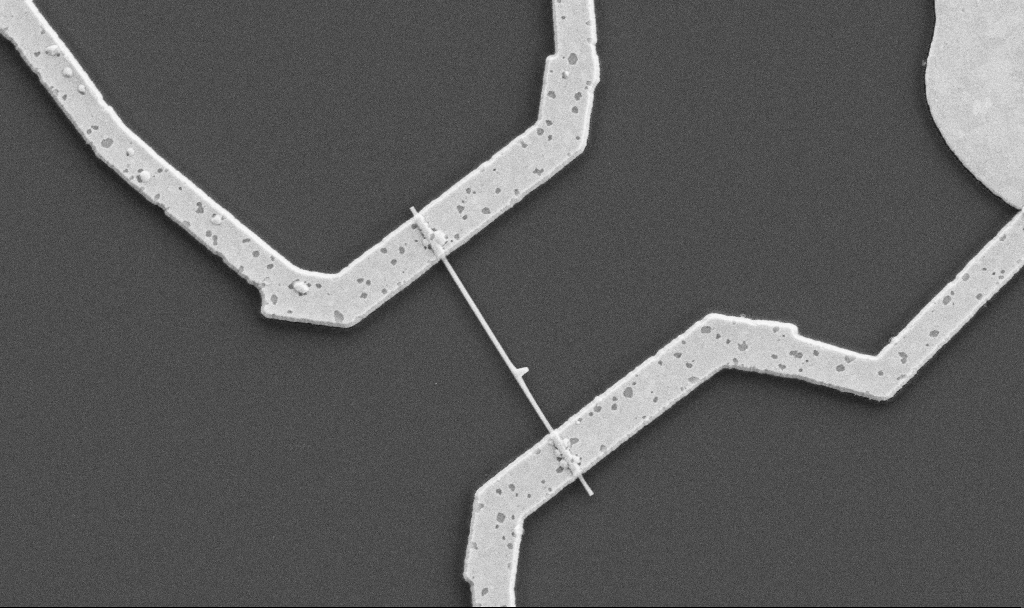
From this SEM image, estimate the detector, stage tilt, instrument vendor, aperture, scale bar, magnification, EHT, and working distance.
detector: SE2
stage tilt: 0°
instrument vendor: Zeiss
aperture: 30 µm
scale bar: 1000 nm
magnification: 20 K X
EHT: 5 kV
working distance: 10.7 mm